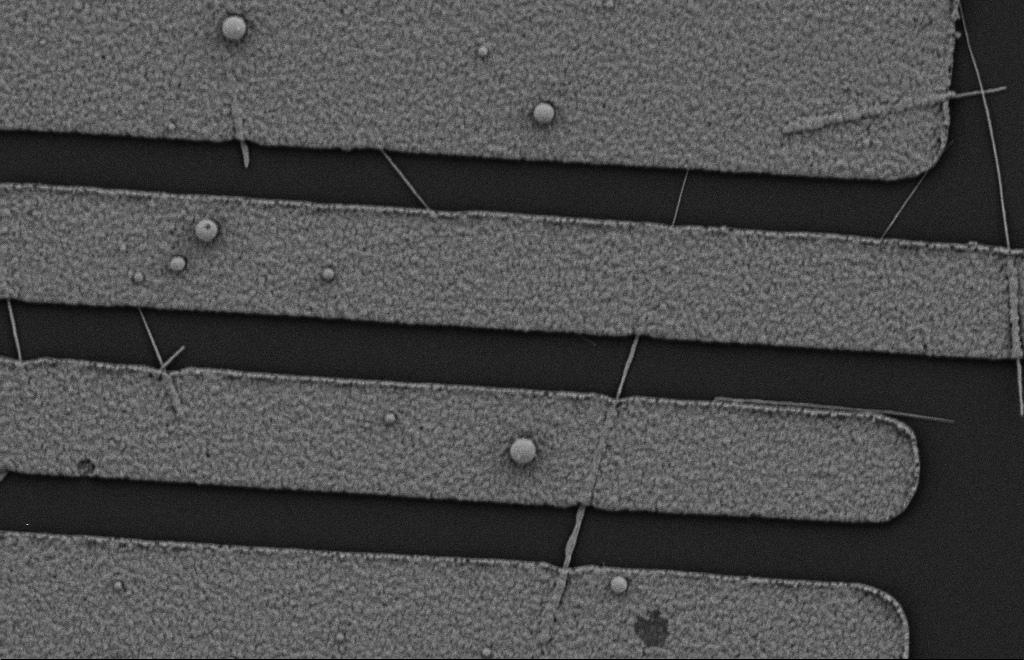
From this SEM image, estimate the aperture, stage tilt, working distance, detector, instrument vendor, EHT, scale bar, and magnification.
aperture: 20 µm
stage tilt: -0.3°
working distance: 10 mm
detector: SE2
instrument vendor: Zeiss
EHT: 2 kV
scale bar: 2000 nm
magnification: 15.8 K X